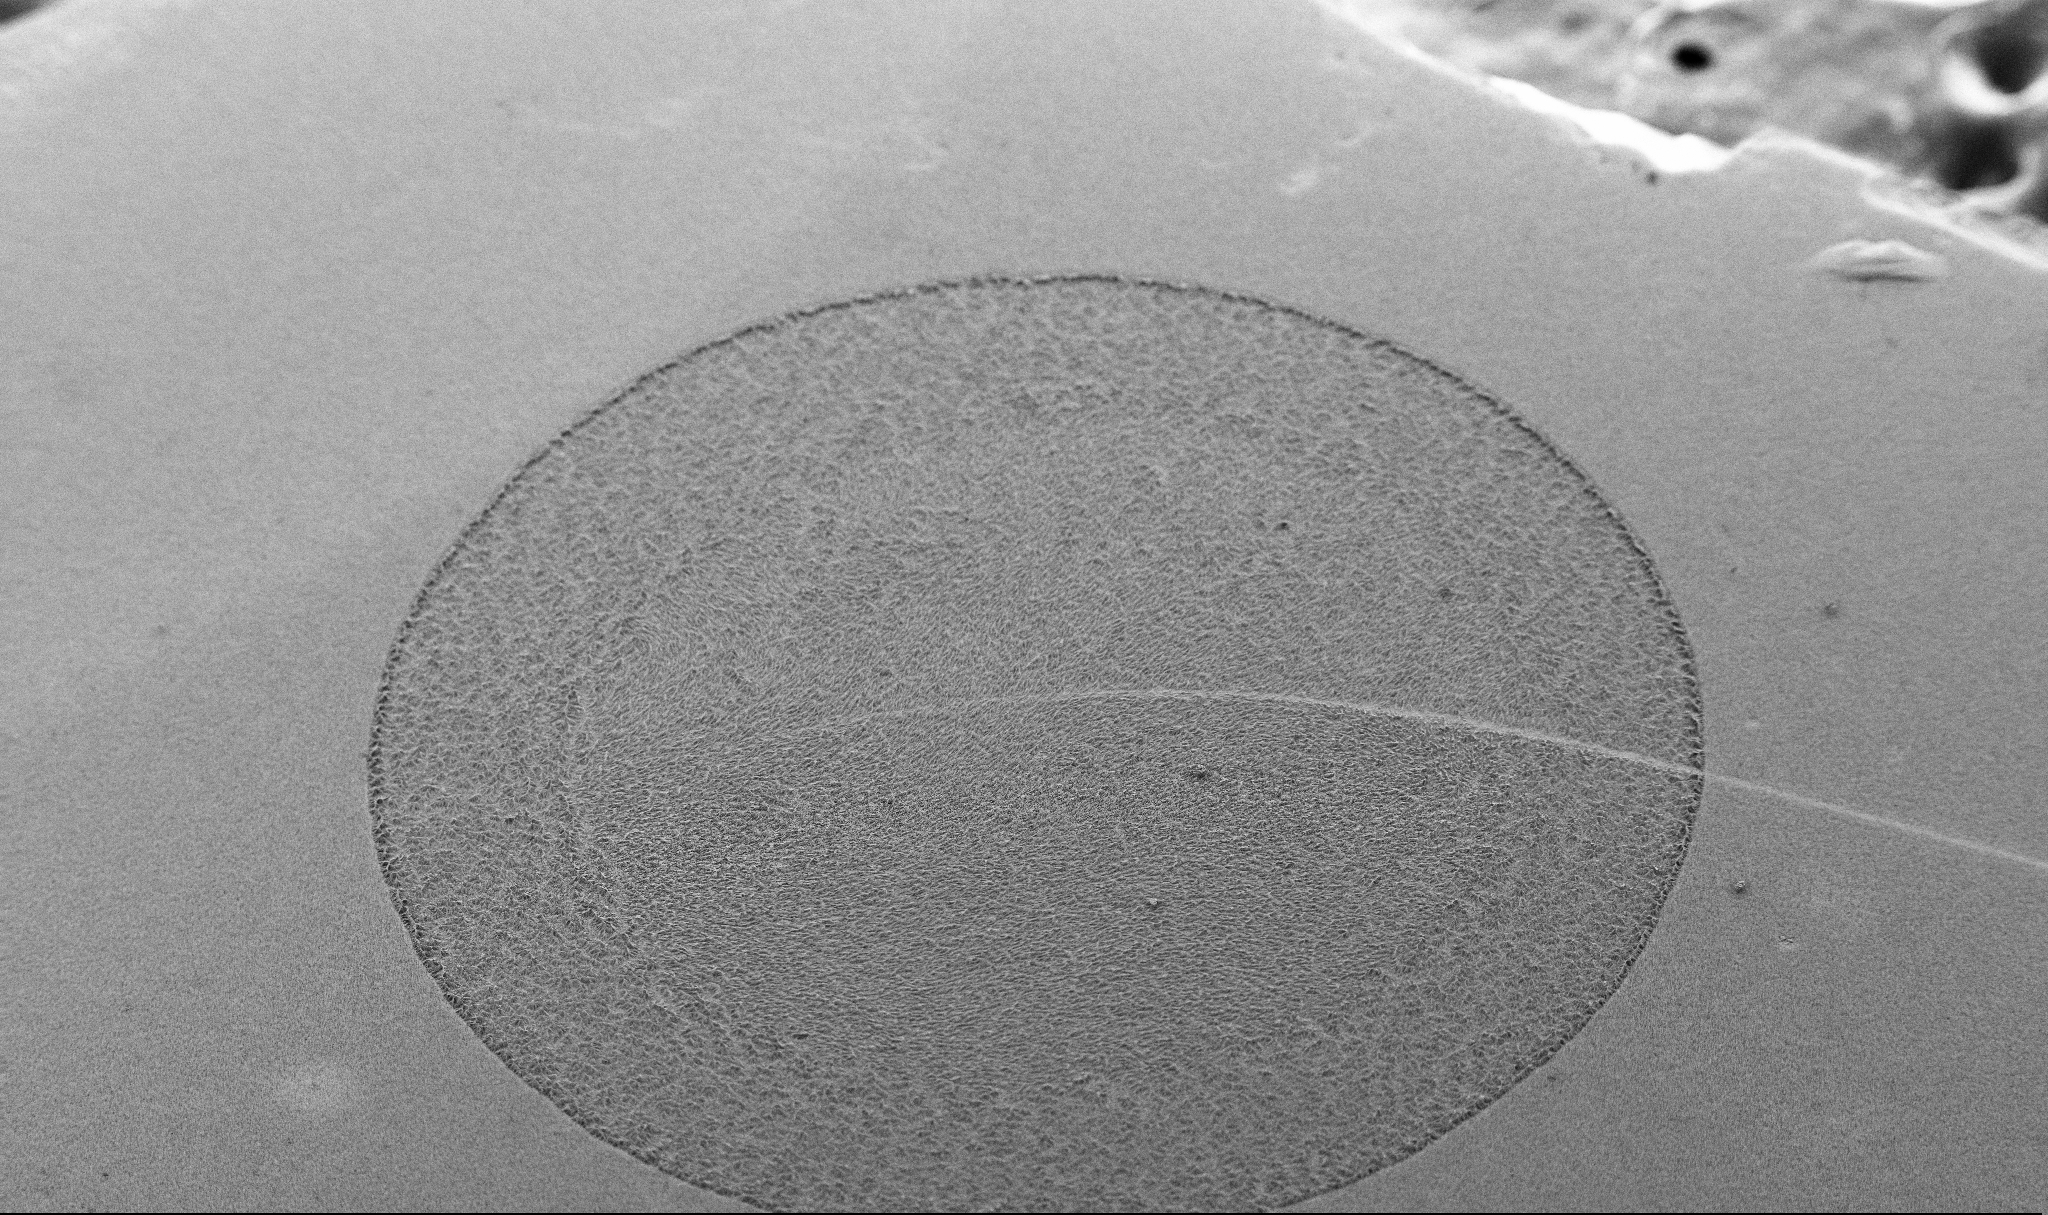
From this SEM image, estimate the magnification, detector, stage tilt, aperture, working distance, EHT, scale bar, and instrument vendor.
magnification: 0.14 K X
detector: SE2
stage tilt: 45°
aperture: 30 µm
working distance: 7.2 mm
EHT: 5 kV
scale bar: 200000 nm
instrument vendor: Zeiss